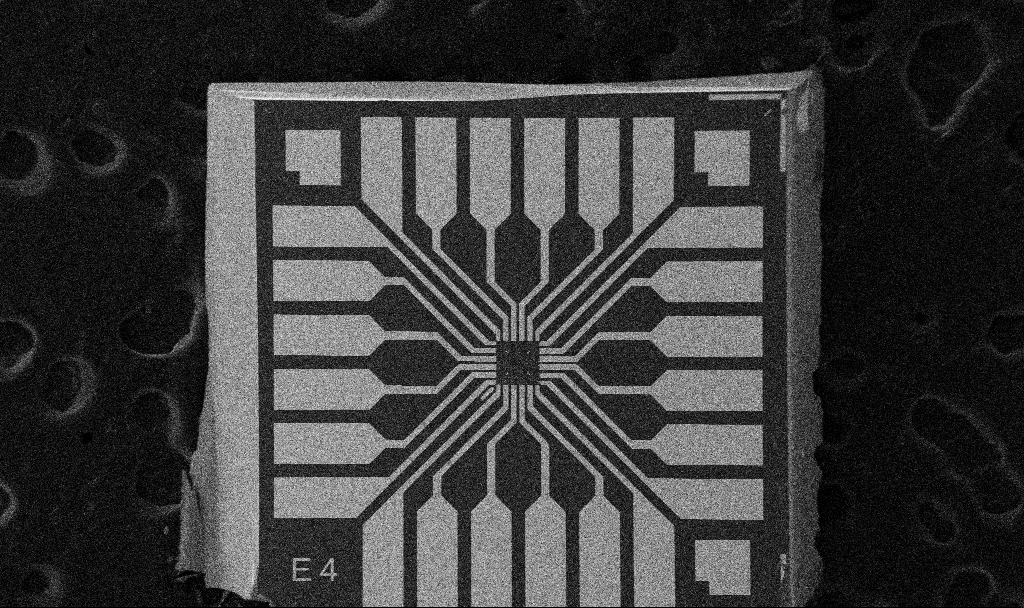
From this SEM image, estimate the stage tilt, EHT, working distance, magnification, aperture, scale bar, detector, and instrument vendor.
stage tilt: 0°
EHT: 5 kV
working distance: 10.7 mm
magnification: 0.1 K X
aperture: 30 µm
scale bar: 200000 nm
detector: SE2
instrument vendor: Zeiss